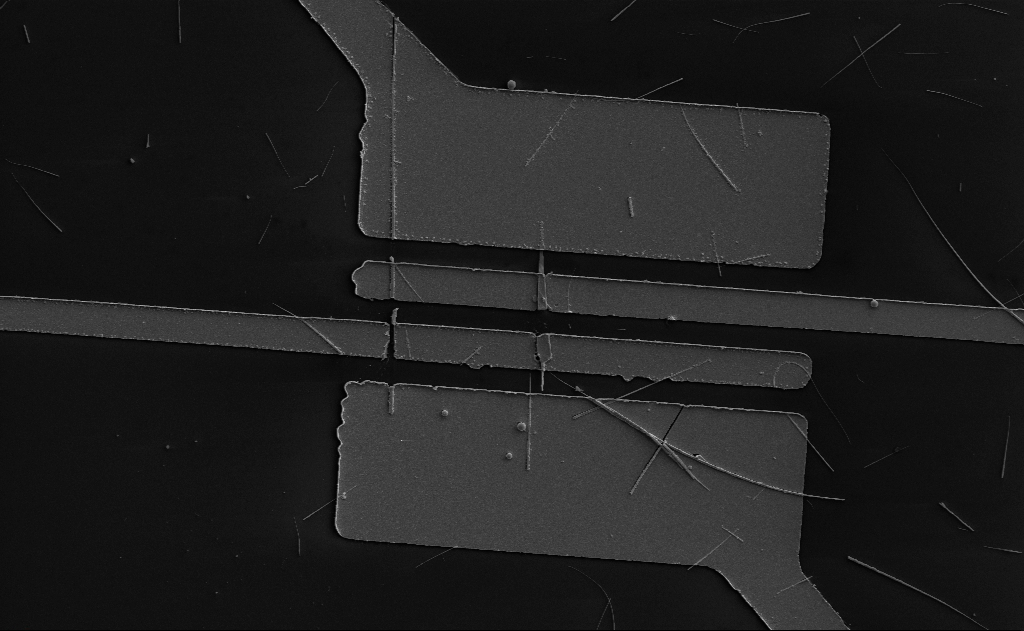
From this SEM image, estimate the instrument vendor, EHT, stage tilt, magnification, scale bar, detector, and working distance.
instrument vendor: Zeiss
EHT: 5 kV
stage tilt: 0°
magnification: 5.68 K X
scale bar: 10000 nm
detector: SE2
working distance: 15 mm